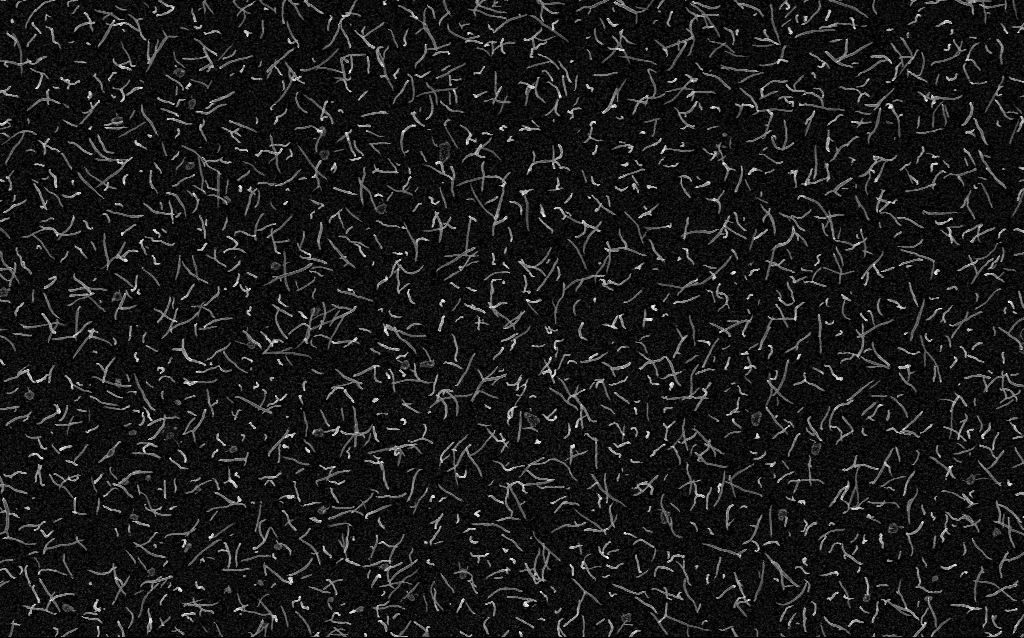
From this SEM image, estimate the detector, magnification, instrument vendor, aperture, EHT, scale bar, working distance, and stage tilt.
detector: InLens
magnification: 10 K X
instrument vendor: Zeiss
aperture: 30 µm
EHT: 5 kV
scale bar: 2000 nm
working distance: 1.5 mm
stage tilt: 0°